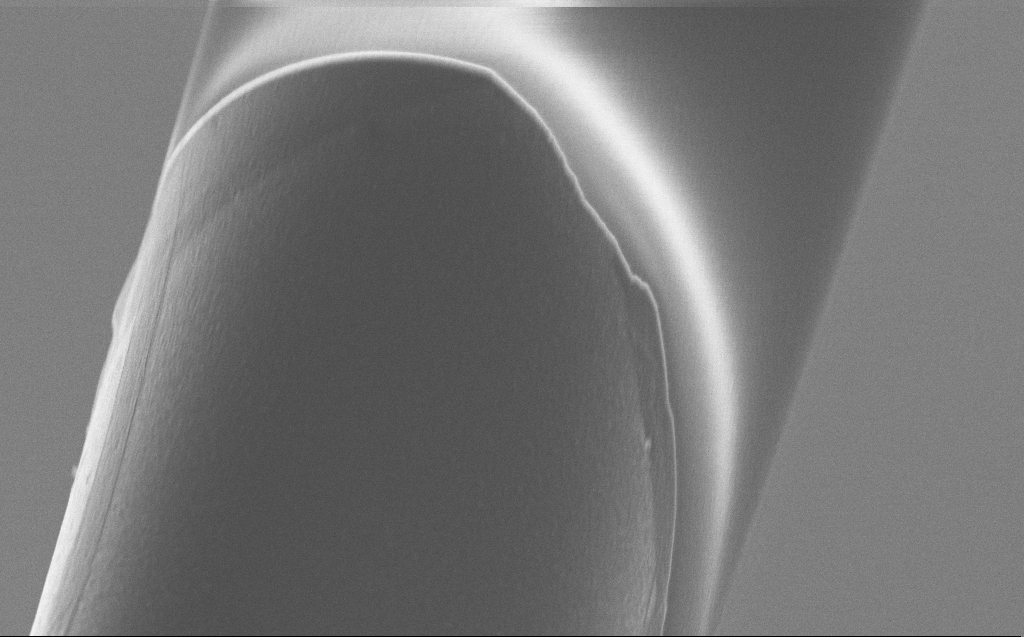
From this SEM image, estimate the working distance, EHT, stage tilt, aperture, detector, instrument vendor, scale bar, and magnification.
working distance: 6 mm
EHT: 5 kV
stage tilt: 45°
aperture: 30 µm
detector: InLens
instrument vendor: Zeiss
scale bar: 2000 nm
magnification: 10 K X